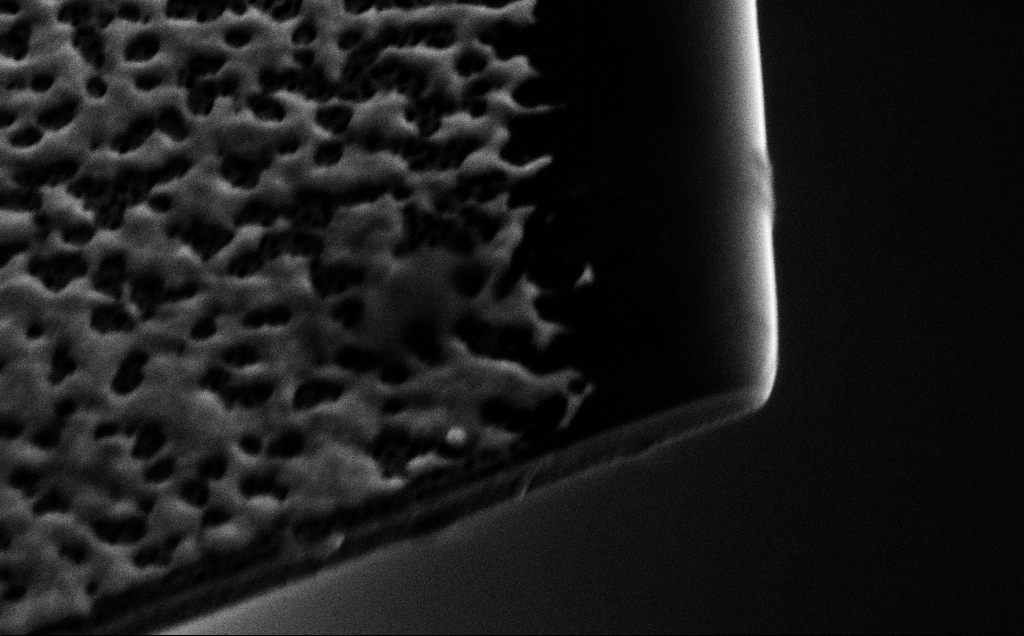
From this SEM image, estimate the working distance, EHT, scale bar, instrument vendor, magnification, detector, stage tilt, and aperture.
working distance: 11 mm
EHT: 10 kV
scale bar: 1000 nm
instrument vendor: Zeiss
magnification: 48.29 K X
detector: SE2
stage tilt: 45°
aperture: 30 µm